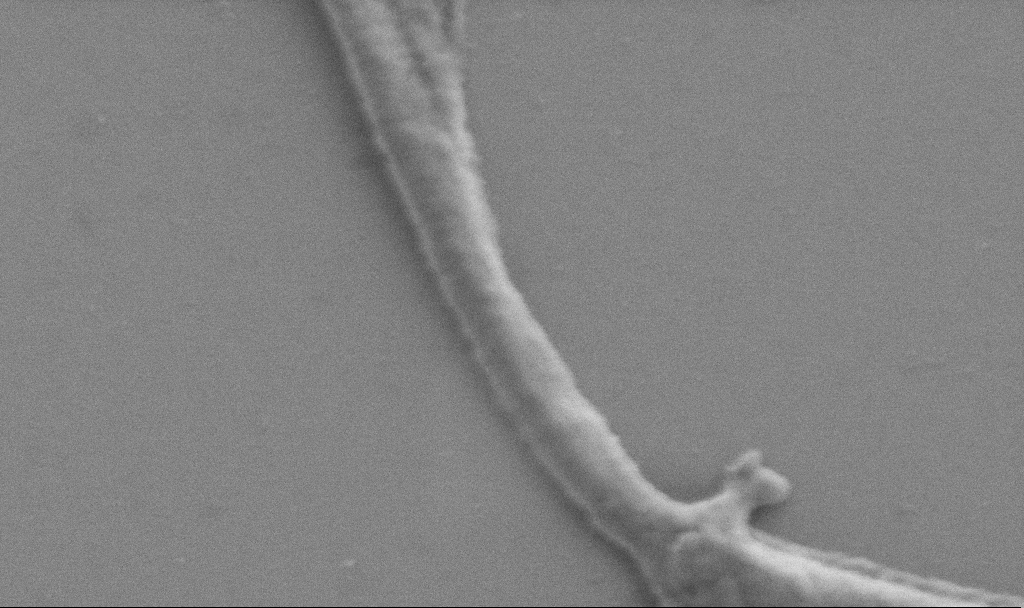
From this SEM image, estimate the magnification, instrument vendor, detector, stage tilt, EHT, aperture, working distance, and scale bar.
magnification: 50 K X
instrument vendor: Zeiss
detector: SE2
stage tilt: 0°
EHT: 0.9 kV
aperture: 30 µm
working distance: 6.9 mm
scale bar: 1000 nm